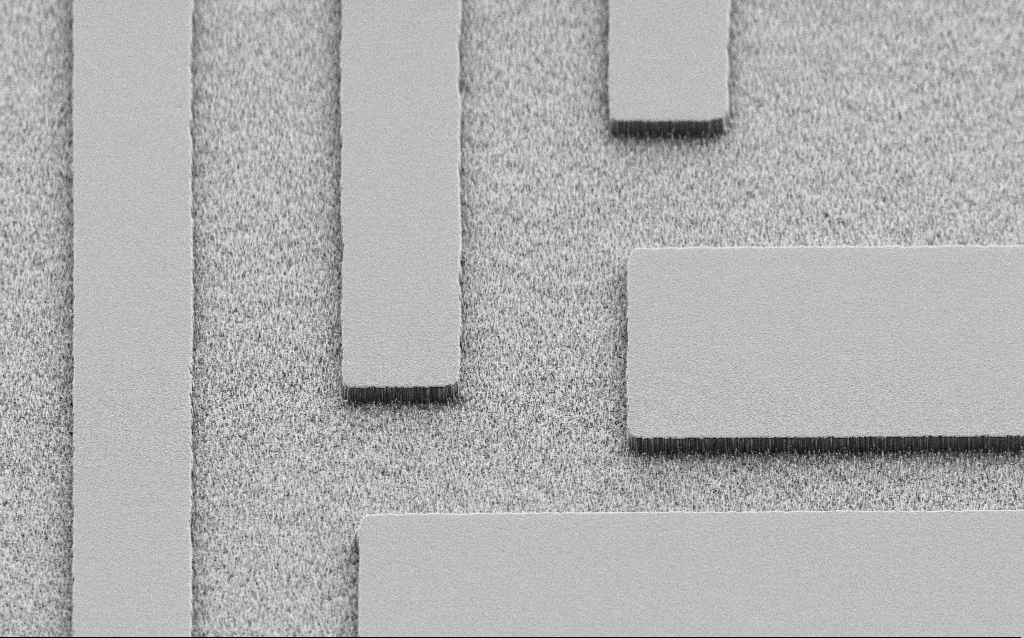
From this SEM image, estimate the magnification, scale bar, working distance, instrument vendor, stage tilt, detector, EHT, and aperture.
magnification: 1.31 K X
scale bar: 20000 nm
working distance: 7 mm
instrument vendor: Zeiss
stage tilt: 45°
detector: SE2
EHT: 2 kV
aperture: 30 µm